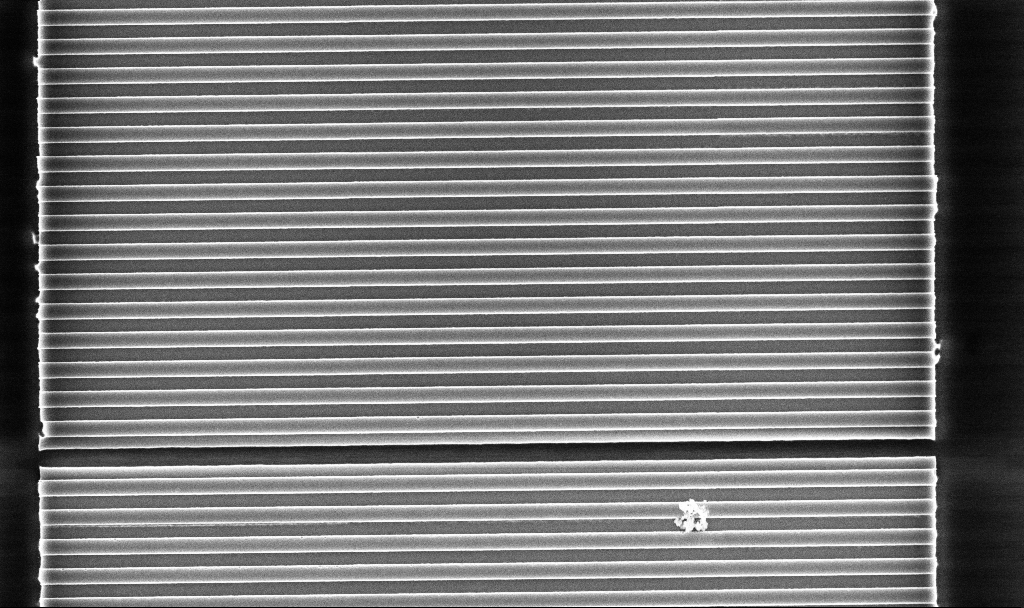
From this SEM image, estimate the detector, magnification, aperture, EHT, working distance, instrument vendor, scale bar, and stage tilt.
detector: InLens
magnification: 16.61 K X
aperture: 30 µm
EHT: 5 kV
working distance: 5.2 mm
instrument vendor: Zeiss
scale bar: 2000 nm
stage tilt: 0°